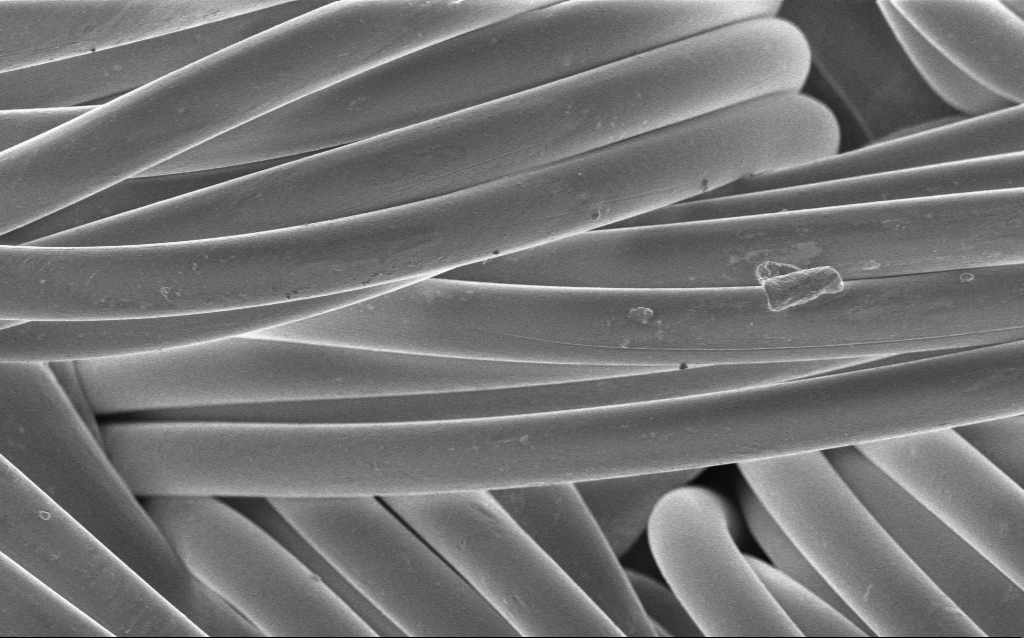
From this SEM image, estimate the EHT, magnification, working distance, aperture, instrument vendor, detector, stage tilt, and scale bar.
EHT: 1 kV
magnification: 1.21 K X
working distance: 4 mm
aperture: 30 µm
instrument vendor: Zeiss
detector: InLens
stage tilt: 0°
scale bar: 20000 nm